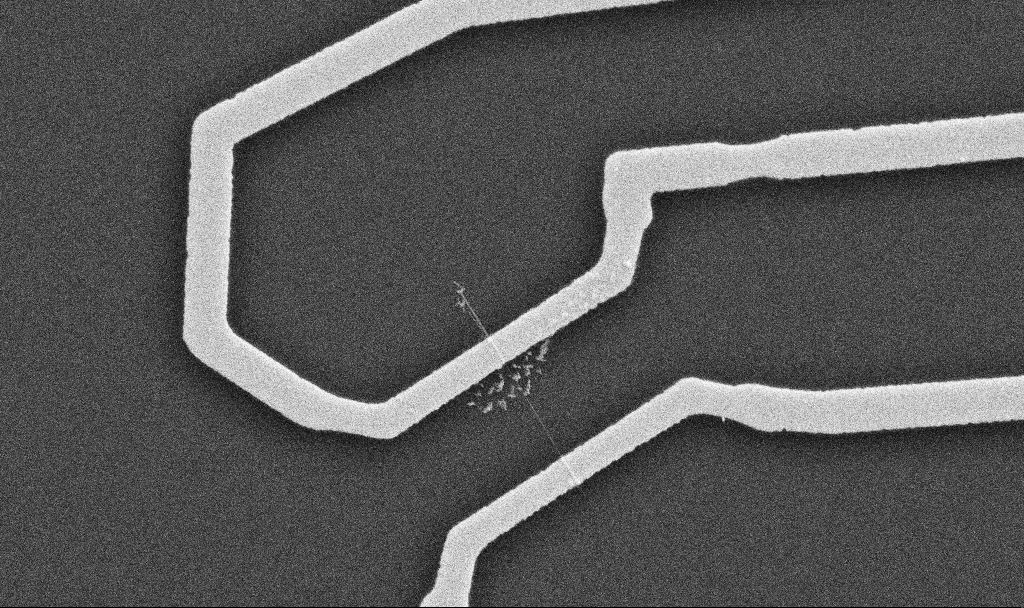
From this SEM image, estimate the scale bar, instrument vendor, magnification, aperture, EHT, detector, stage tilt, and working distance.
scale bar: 1000 nm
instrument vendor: Zeiss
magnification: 20 K X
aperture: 30 µm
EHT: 10 kV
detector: SE2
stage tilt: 0°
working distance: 10.7 mm